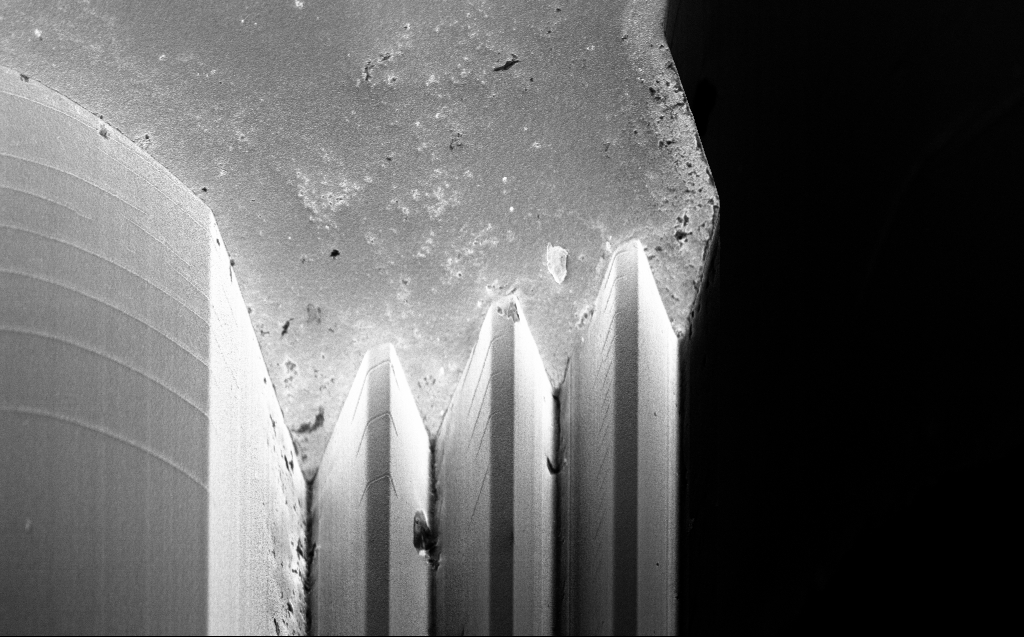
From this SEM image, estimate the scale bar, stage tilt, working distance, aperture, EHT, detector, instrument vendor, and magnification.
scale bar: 10000 nm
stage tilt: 45°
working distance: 8 mm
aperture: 30 µm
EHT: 3 kV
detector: InLens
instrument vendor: Zeiss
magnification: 4.22 K X